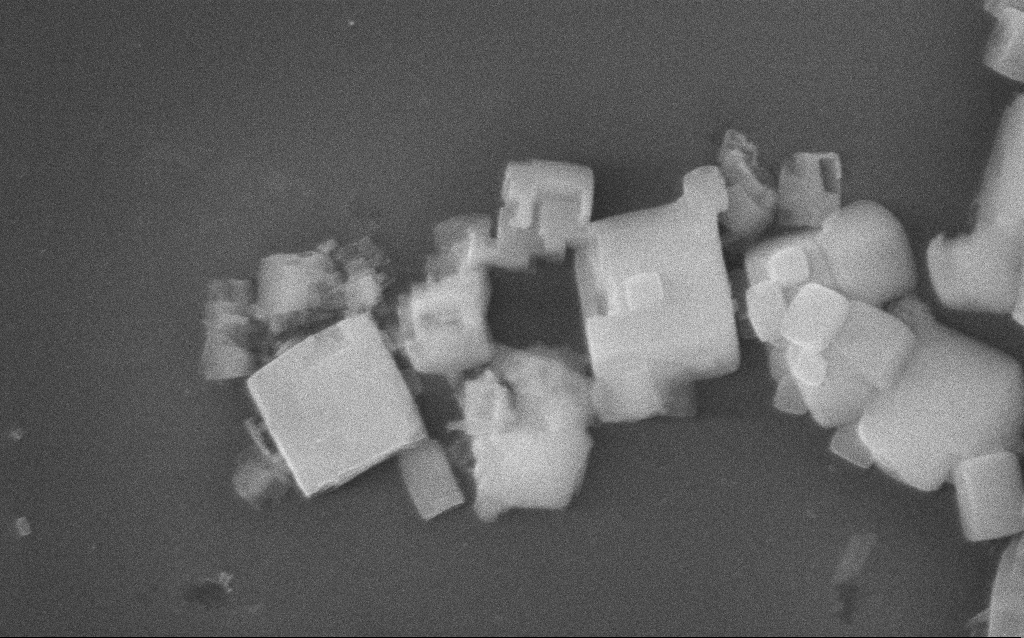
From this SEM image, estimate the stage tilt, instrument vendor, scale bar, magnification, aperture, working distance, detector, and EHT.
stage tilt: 0°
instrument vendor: Zeiss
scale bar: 1000 nm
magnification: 57.28 K X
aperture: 30 µm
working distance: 2 mm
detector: InLens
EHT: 10 kV